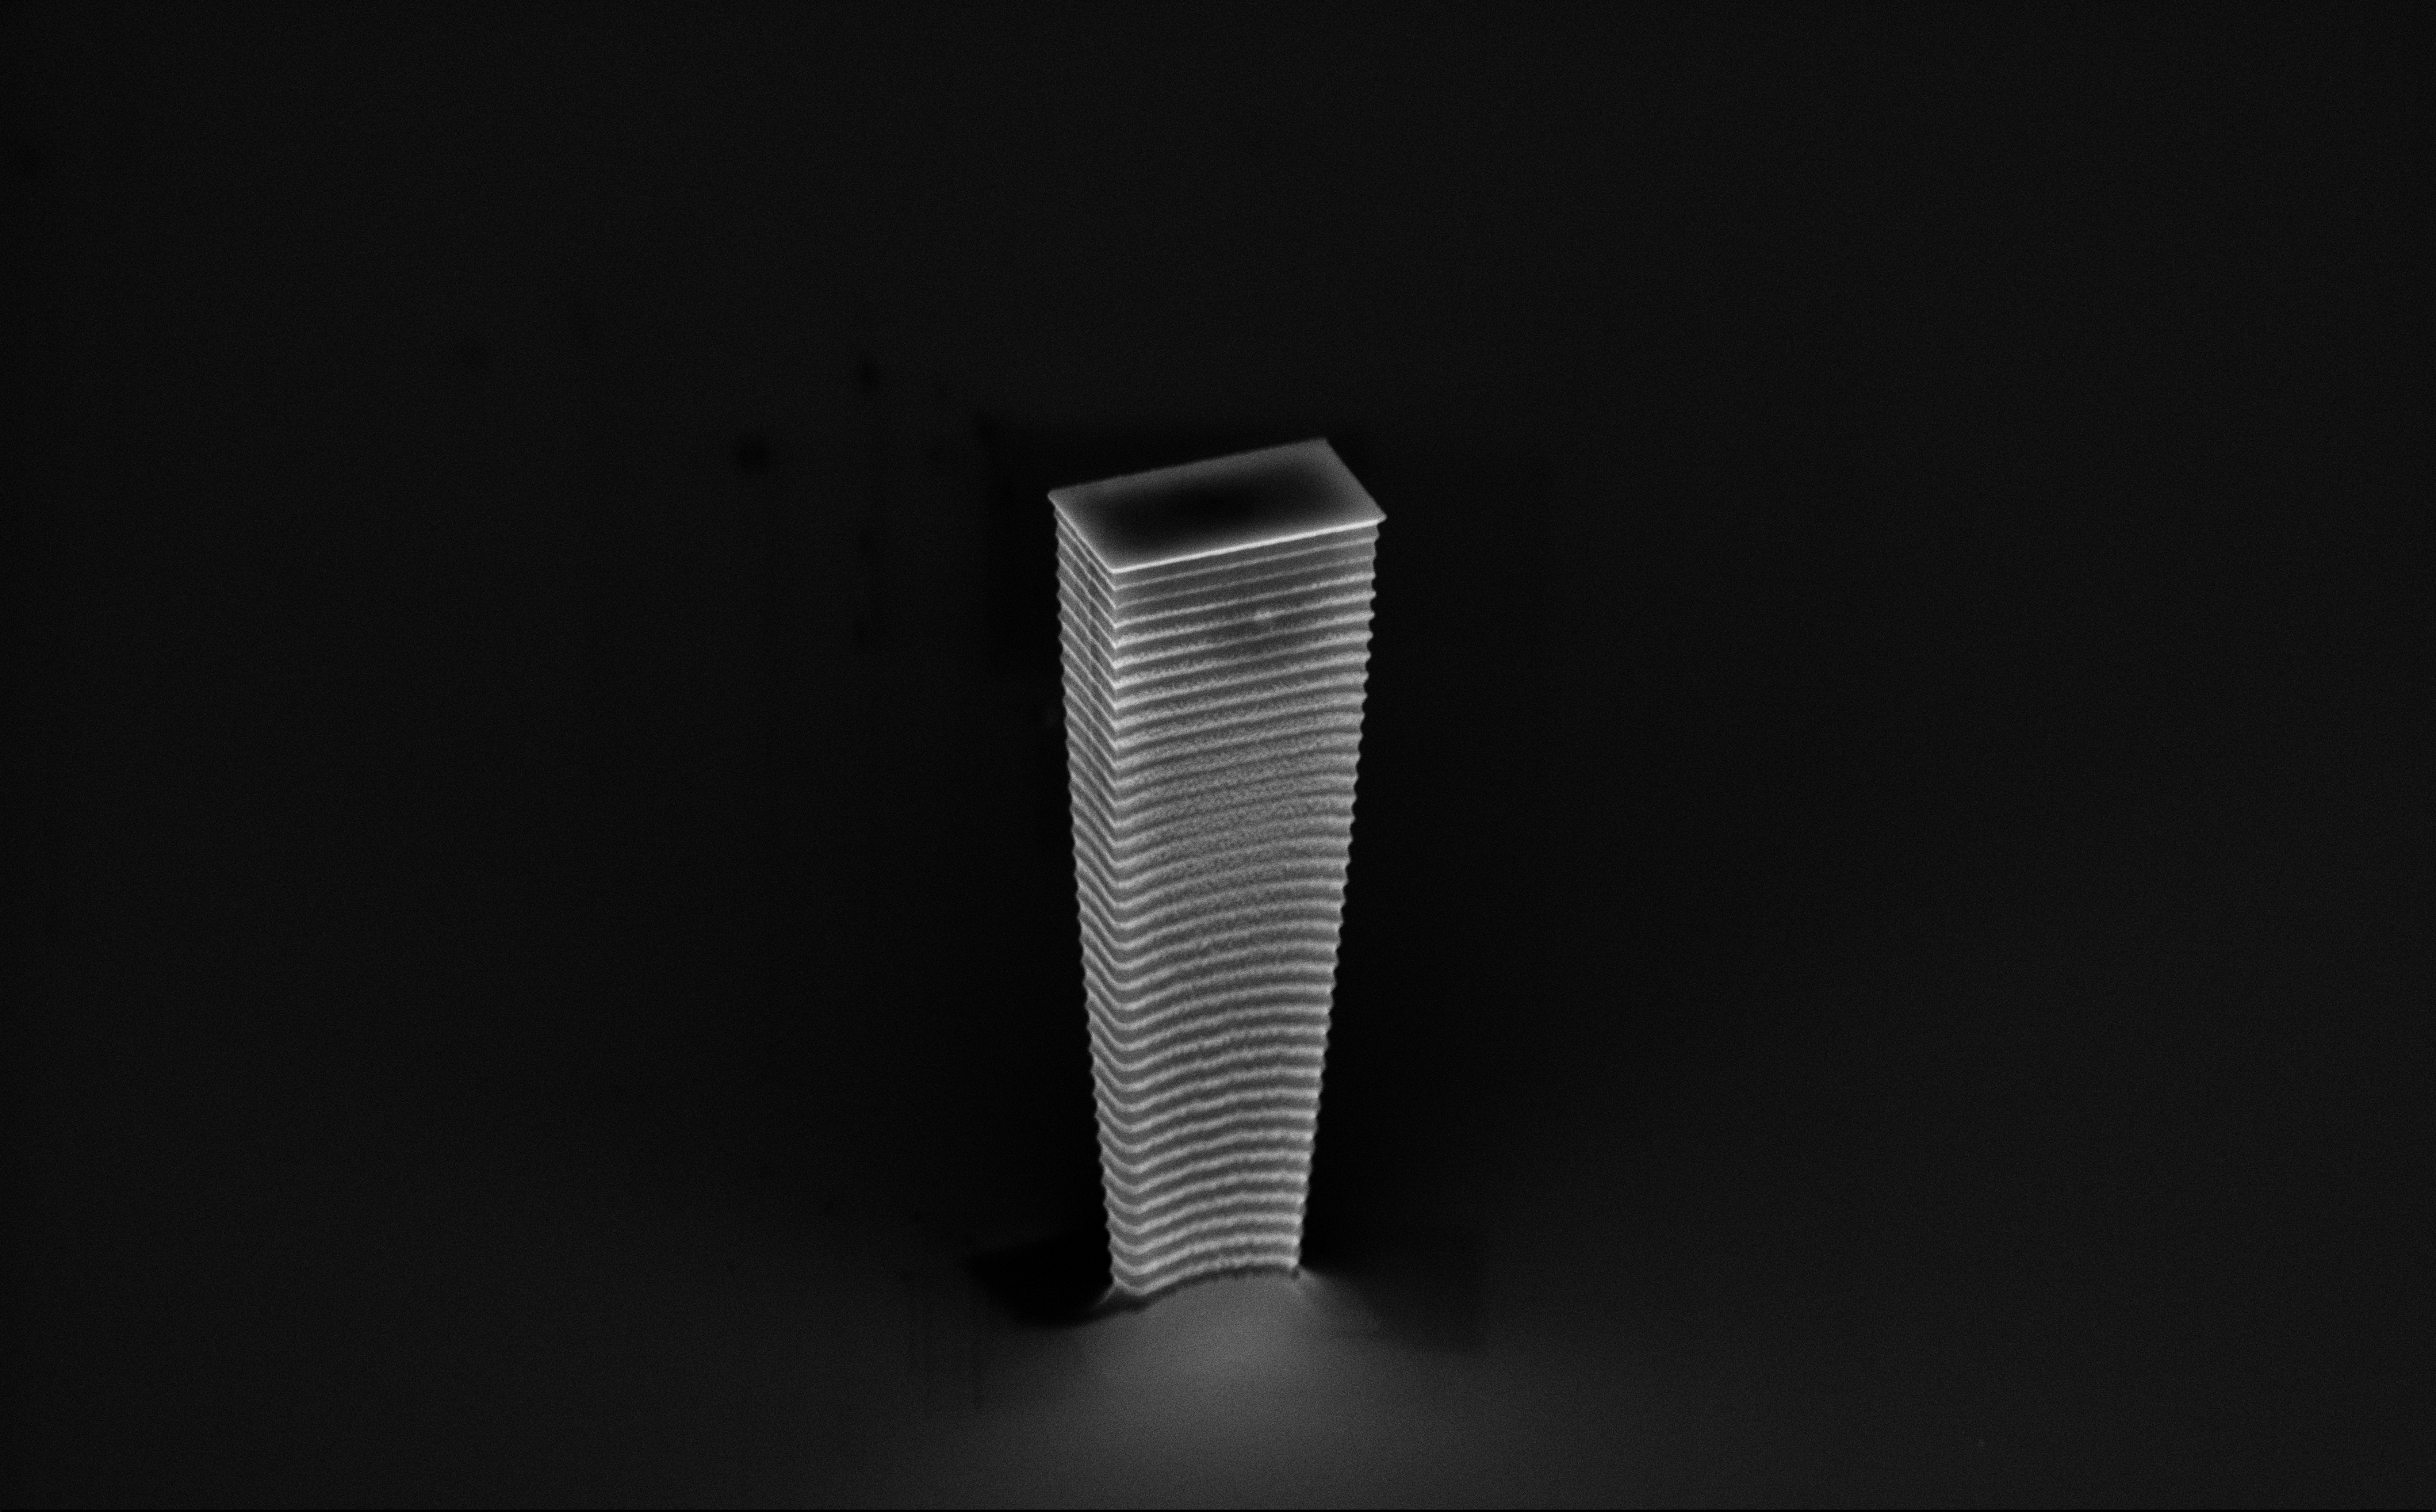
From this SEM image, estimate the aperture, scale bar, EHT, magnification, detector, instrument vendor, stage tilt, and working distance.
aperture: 30 µm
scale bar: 2000 nm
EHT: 10 kV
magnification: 9.69 K X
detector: InLens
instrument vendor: Zeiss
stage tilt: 60°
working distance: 5 mm